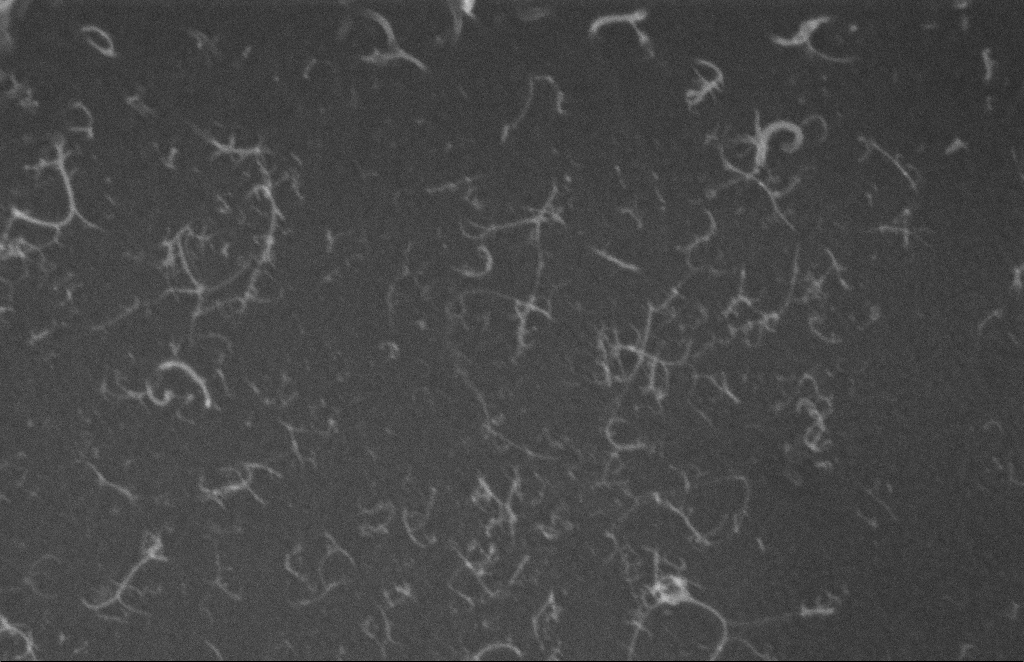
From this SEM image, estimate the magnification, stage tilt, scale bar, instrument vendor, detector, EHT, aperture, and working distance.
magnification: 212.89 K X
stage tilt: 0°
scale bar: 200 nm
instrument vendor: Zeiss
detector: InLens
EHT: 5 kV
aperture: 10 µm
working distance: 8 mm